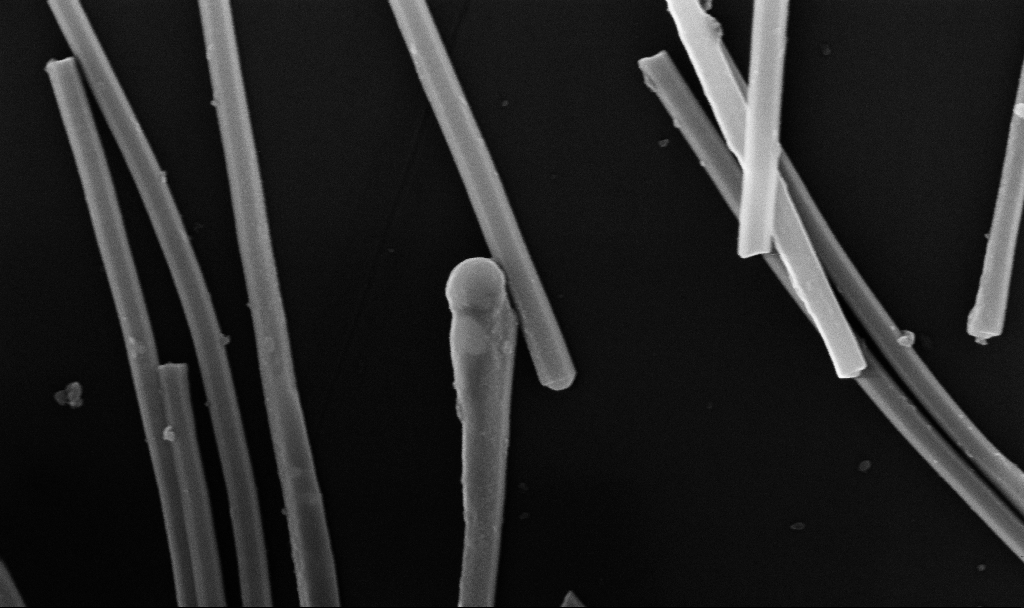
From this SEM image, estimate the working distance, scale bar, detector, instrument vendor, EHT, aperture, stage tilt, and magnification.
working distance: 6.7 mm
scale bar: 200 nm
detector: InLens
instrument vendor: Zeiss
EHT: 10 kV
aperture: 30 µm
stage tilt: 0°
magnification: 104.61 K X